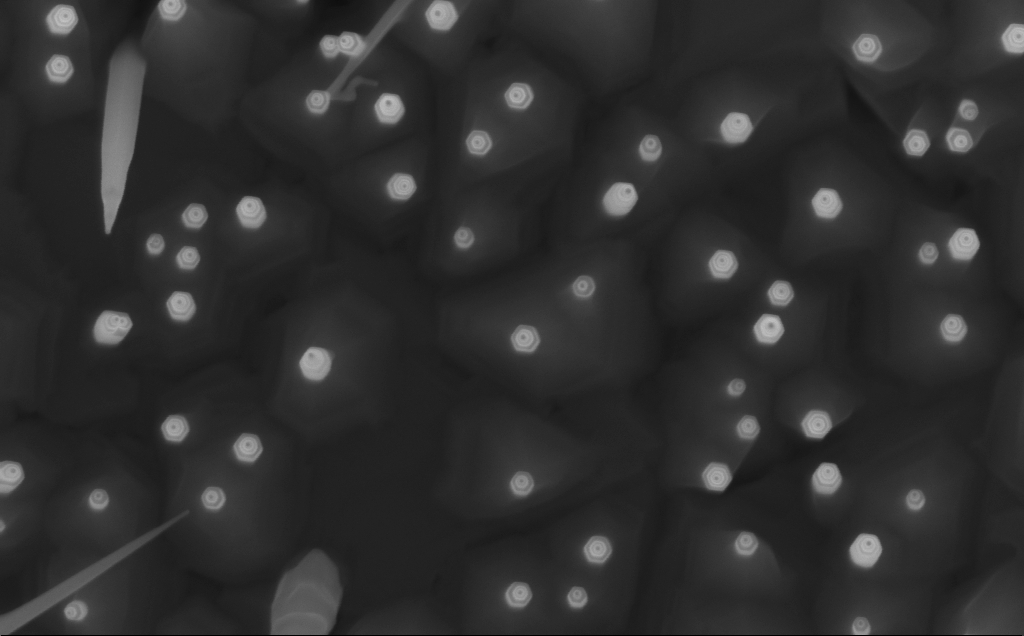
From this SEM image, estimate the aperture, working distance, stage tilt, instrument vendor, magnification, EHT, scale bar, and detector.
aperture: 30 µm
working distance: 5 mm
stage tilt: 0°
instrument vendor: Zeiss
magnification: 100 K X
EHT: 10 kV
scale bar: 200 nm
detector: InLens